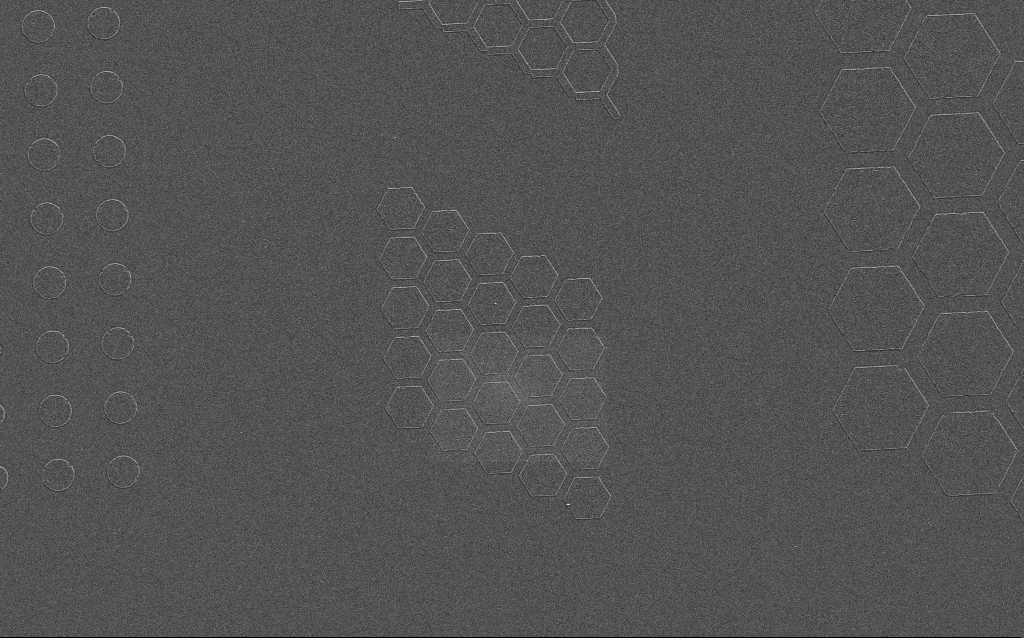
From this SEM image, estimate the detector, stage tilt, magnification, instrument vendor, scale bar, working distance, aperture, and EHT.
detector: SE2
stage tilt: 0°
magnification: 5.89 K X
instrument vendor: Zeiss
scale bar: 10000 nm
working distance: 7 mm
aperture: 30 µm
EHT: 1.5 kV